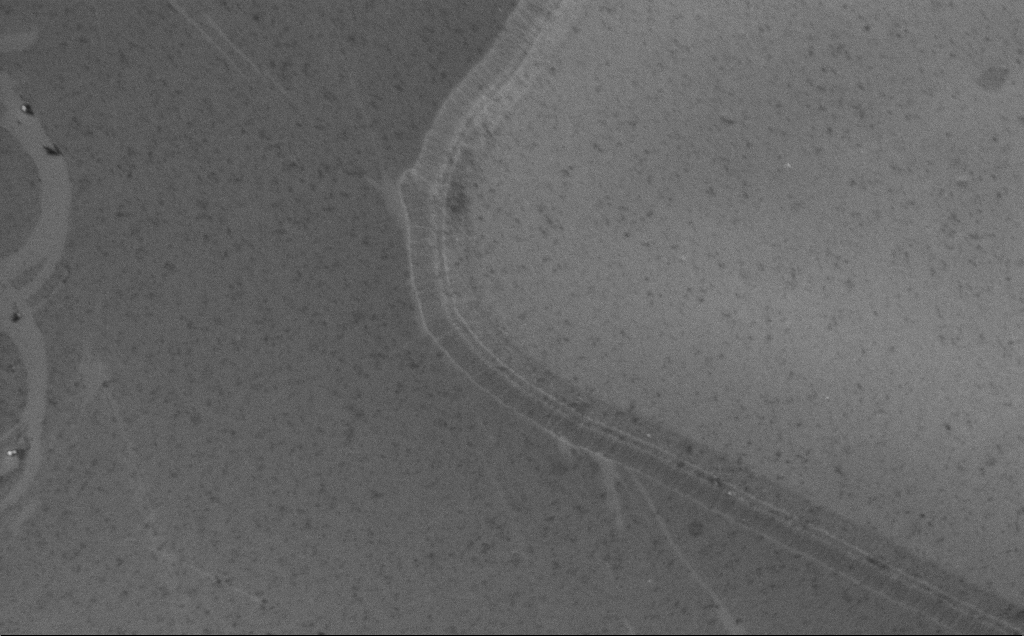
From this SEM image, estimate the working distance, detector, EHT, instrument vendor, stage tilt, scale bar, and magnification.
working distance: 8 mm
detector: InLens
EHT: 5 kV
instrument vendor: Zeiss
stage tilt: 30°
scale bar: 2000 nm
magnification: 16.17 K X